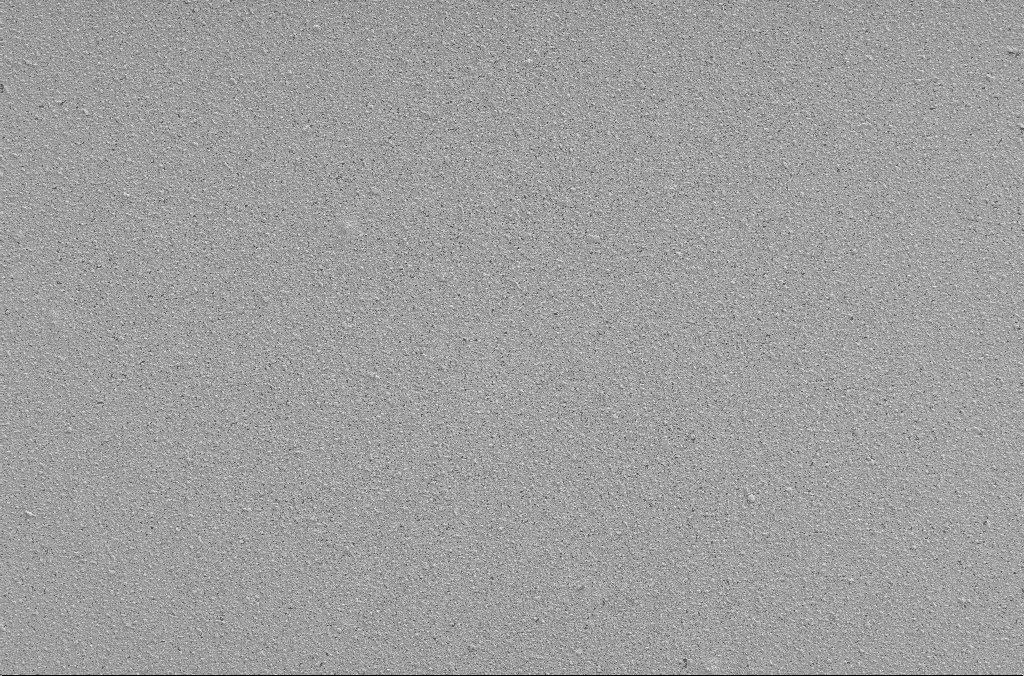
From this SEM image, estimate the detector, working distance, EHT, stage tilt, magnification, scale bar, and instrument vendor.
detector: SE2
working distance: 3 mm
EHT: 2 kV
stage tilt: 0°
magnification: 1 K X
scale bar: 20000 nm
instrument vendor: Zeiss